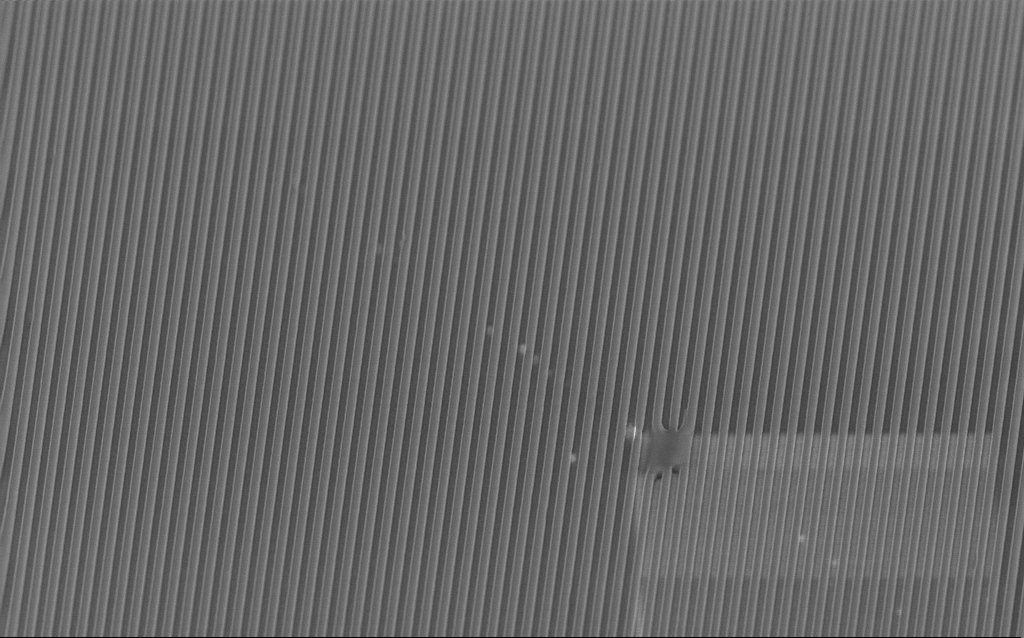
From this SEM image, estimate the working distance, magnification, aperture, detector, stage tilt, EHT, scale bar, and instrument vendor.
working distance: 3.6 mm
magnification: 12.52 K X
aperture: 30 µm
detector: InLens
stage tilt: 45°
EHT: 2 kV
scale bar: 1000 nm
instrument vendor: Zeiss